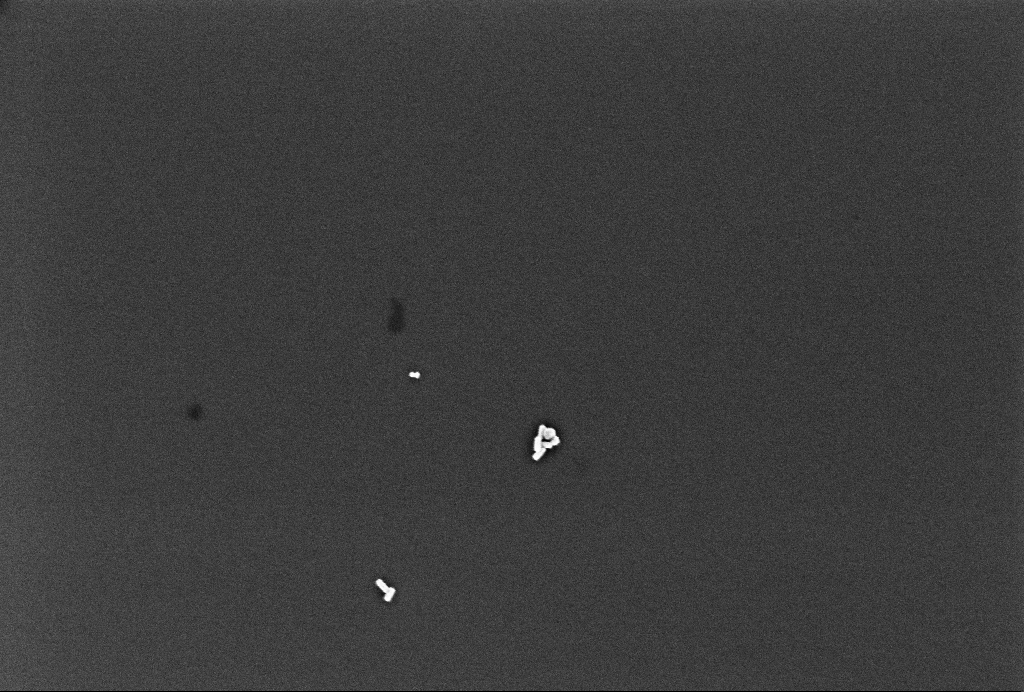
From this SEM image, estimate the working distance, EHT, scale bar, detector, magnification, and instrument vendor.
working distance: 3.3 mm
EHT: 2 kV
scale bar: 200 nm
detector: InLens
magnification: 77.16 K X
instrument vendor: Zeiss